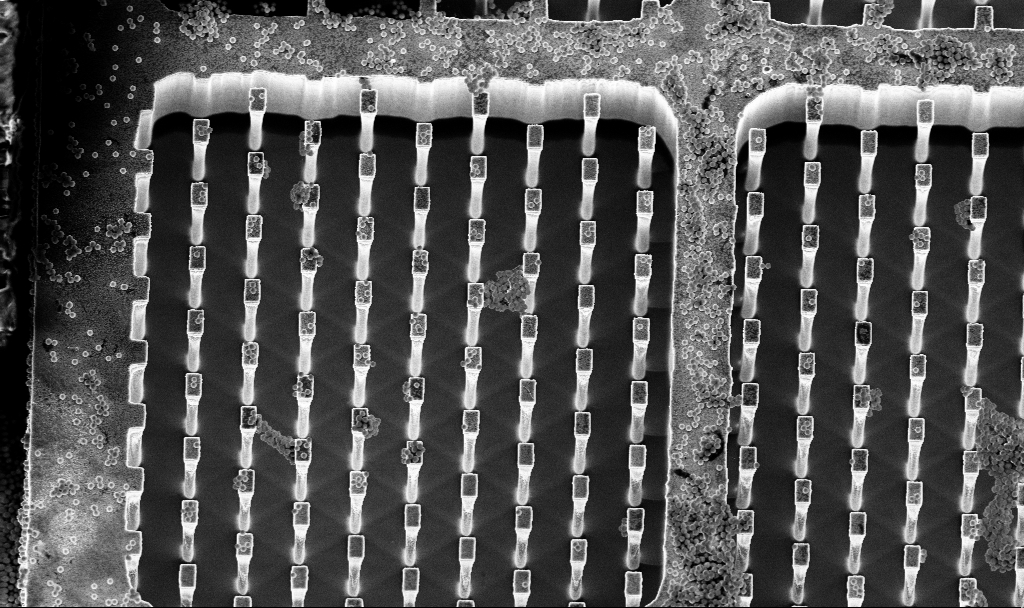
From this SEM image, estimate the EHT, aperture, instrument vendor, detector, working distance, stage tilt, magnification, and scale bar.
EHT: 5 kV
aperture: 30 µm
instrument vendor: Zeiss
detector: InLens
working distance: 3.2 mm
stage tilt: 15°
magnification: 3.74 K X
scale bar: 10000 nm